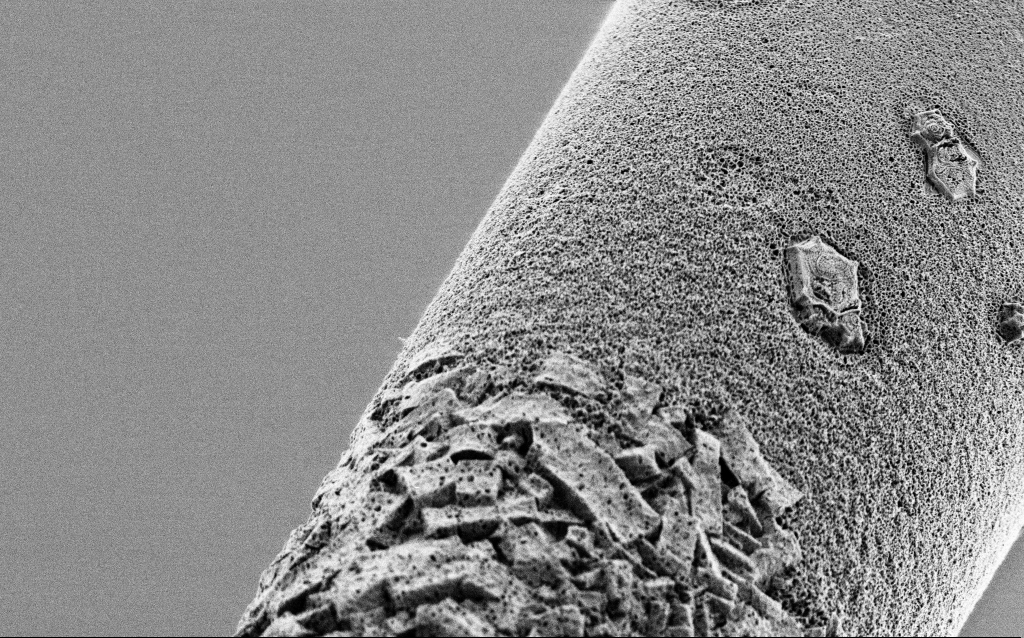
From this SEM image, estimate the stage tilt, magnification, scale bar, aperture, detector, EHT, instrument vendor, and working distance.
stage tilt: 45°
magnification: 10 K X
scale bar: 2000 nm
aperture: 30 µm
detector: SE2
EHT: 1 kV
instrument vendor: Zeiss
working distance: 6.8 mm